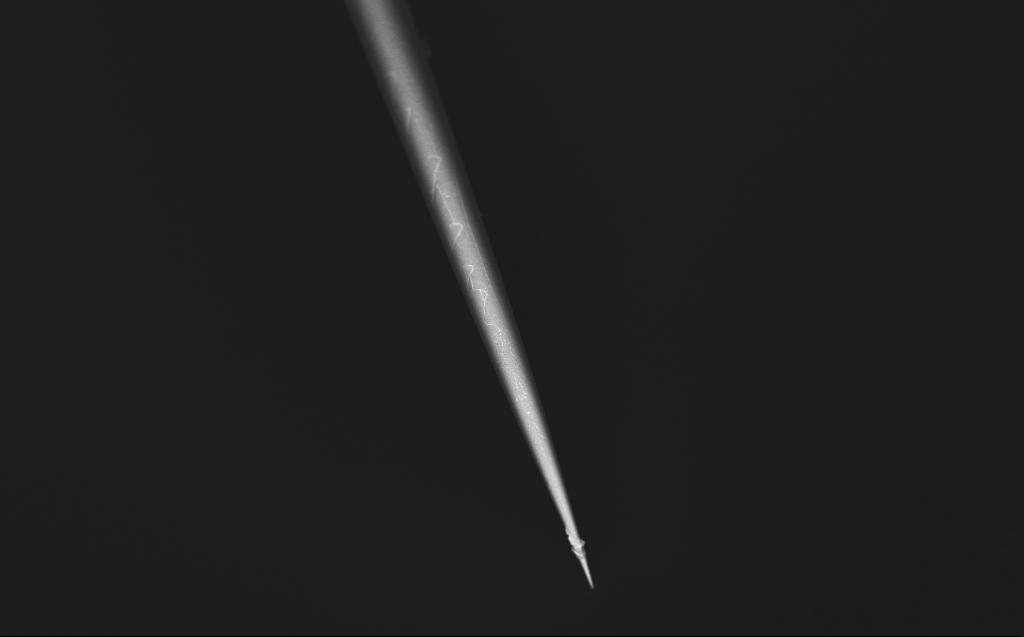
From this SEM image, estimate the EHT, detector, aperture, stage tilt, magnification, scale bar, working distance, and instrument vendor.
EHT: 1 kV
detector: InLens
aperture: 30 µm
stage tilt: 45°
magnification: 1 K X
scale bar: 20000 nm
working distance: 4 mm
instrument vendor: Zeiss